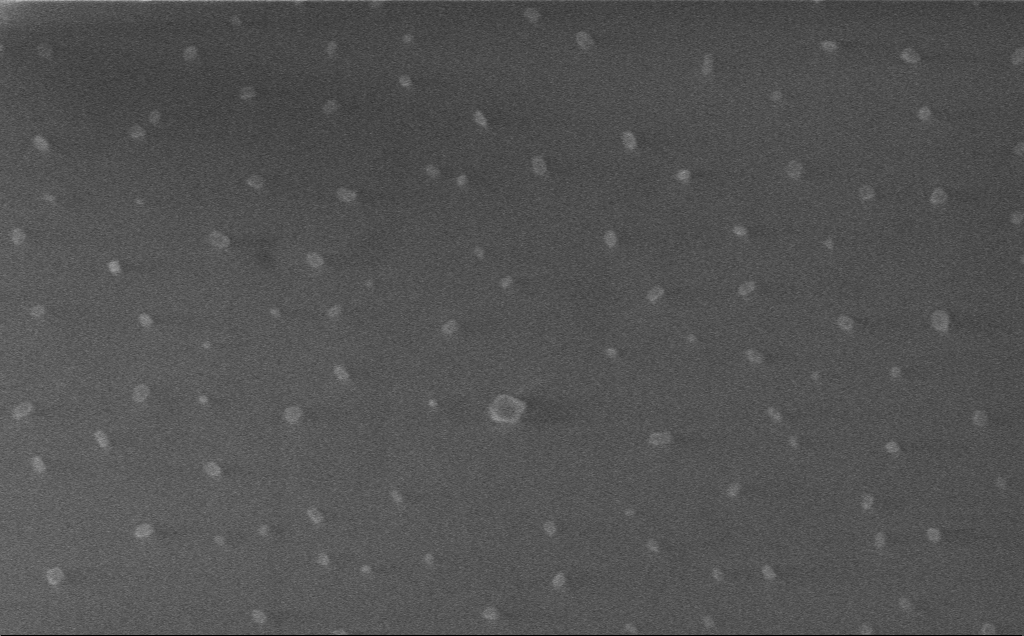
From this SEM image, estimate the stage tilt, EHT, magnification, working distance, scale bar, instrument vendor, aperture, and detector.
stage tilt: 0°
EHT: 1 kV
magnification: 76.43 K X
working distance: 3 mm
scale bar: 200 nm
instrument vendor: Zeiss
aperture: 30 µm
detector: InLens